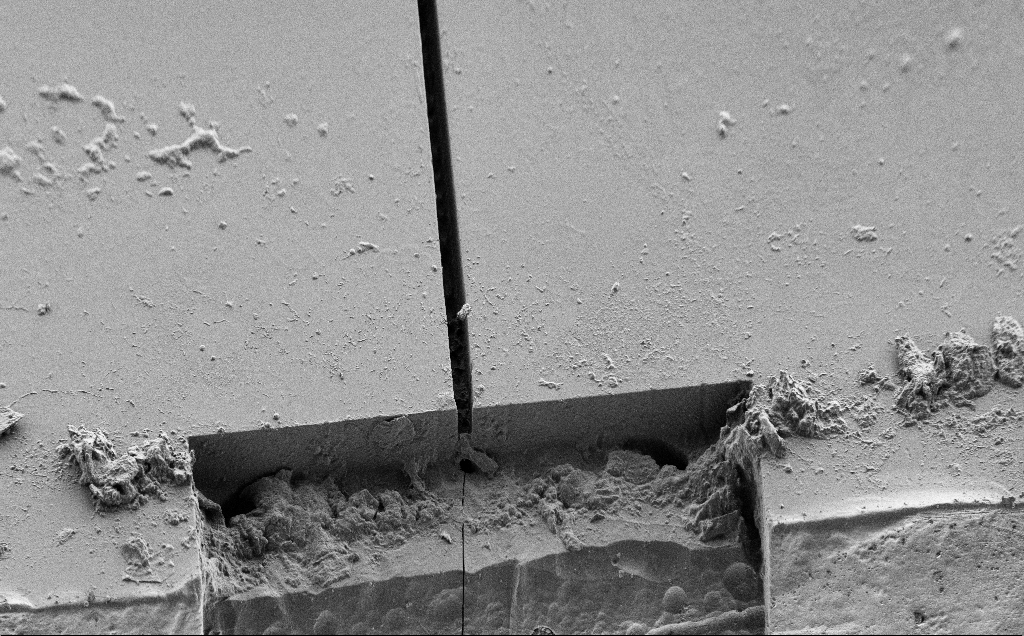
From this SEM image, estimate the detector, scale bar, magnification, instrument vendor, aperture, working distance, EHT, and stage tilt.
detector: SE2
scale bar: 100000 nm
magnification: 0.512 K X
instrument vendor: Zeiss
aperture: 30 µm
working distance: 6 mm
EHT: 1 kV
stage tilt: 45°